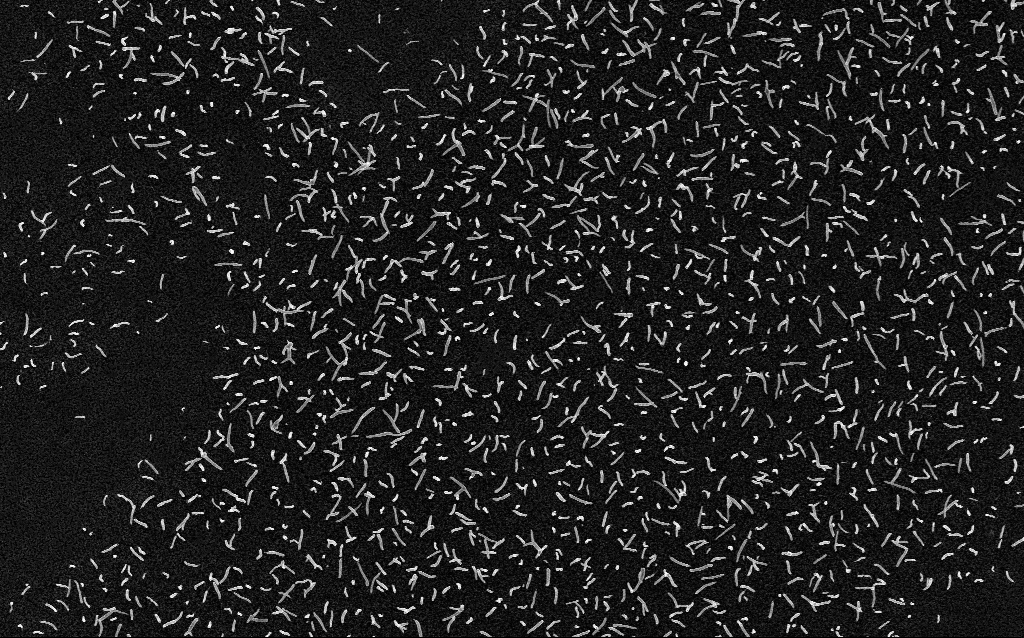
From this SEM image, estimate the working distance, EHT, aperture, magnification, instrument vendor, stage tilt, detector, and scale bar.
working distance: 1.8 mm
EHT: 5 kV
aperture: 30 µm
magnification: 10 K X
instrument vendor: Zeiss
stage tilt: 0°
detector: InLens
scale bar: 2000 nm